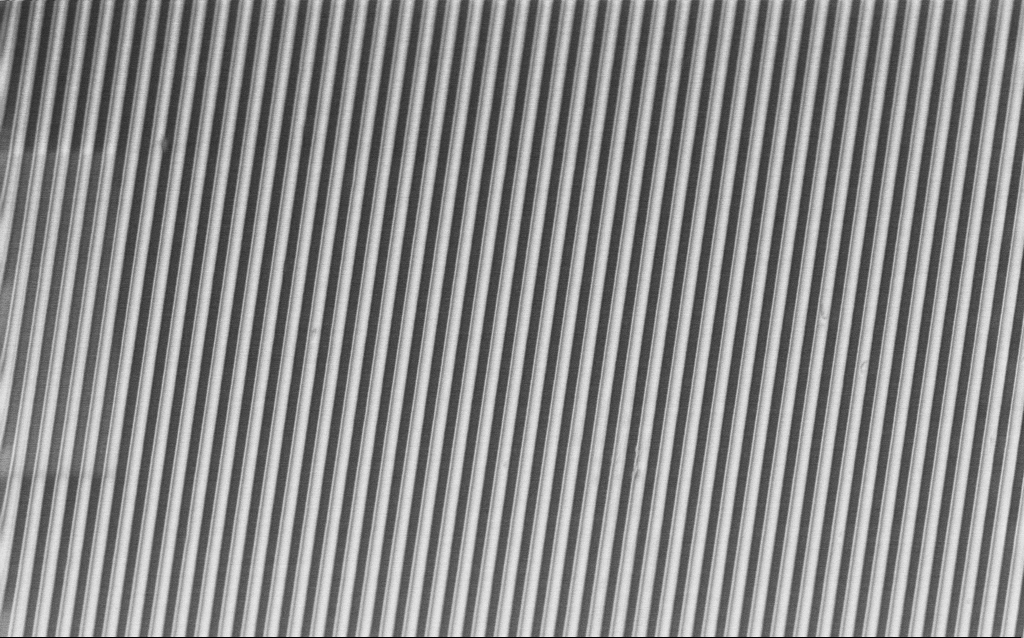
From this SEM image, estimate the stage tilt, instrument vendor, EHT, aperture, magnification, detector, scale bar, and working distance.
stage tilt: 45°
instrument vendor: Zeiss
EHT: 2 kV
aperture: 30 µm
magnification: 16.92 K X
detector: InLens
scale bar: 2000 nm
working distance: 4.1 mm